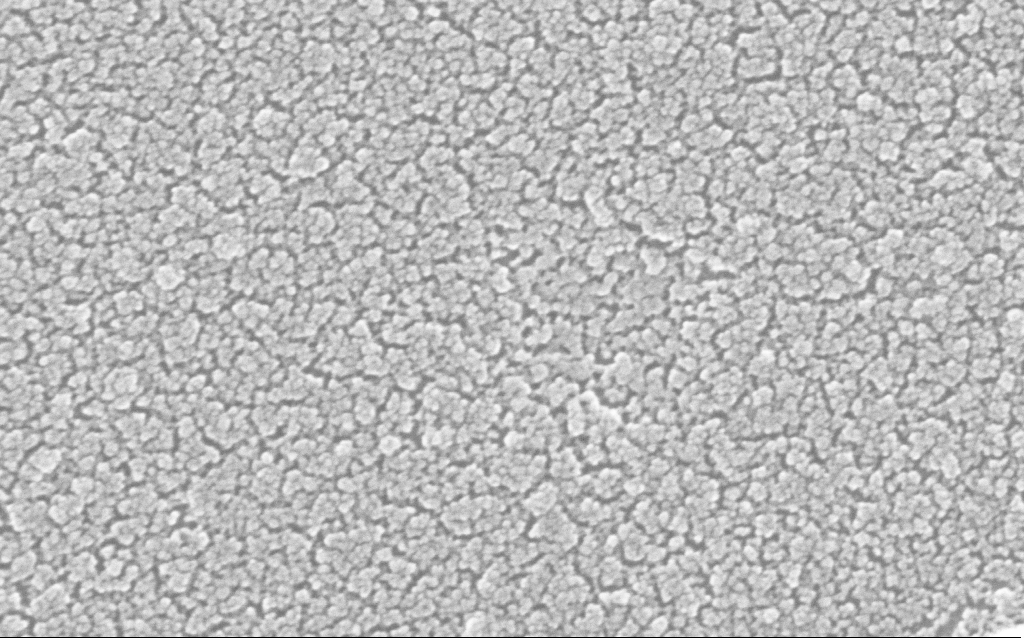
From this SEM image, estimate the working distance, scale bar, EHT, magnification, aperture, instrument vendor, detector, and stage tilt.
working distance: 1.8 mm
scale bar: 100 nm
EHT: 20 kV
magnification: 500 K X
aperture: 30 µm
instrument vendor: Zeiss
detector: InLens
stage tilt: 0°